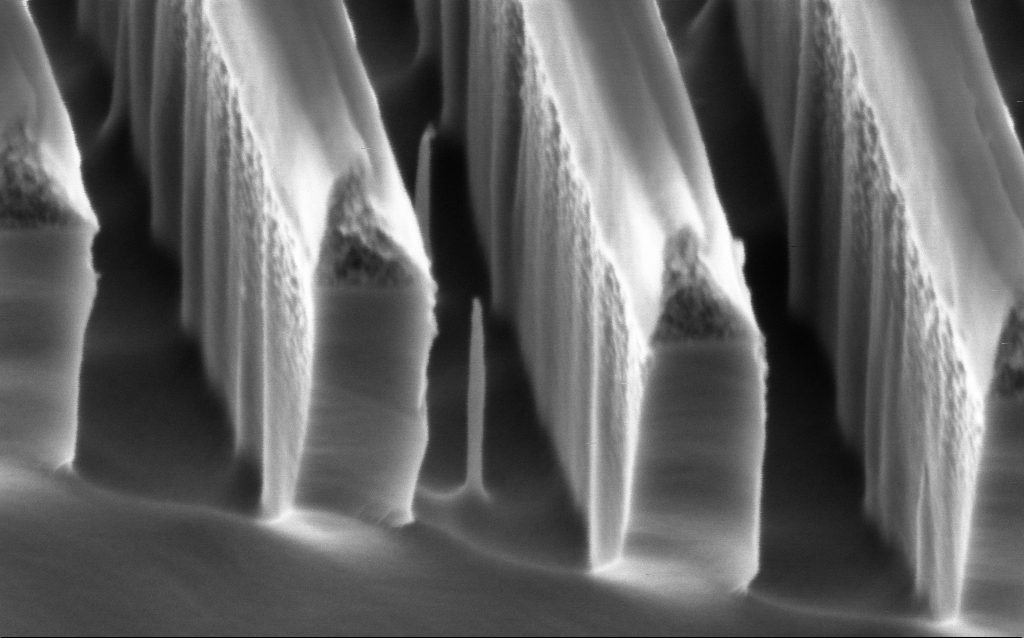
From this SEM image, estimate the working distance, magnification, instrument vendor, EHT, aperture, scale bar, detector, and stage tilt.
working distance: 3.6 mm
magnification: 220.73 K X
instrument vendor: Zeiss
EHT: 2 kV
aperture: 30 µm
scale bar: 200 nm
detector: InLens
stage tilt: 45°